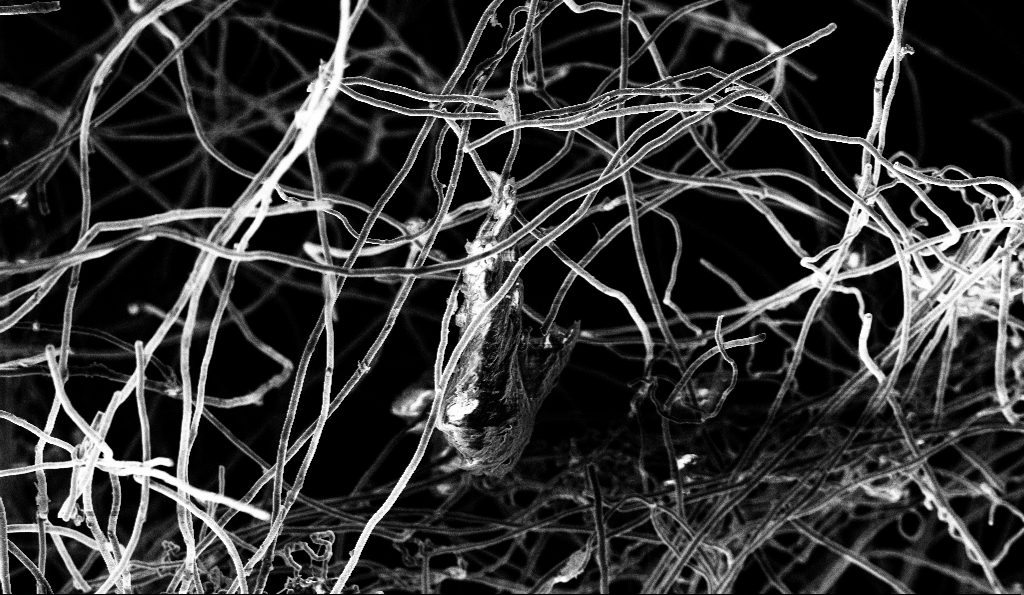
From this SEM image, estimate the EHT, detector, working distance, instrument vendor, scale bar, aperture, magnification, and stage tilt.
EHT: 3 kV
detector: InLens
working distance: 5.9 mm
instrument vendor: Zeiss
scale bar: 10000 nm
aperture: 30 µm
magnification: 5 K X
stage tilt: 0°